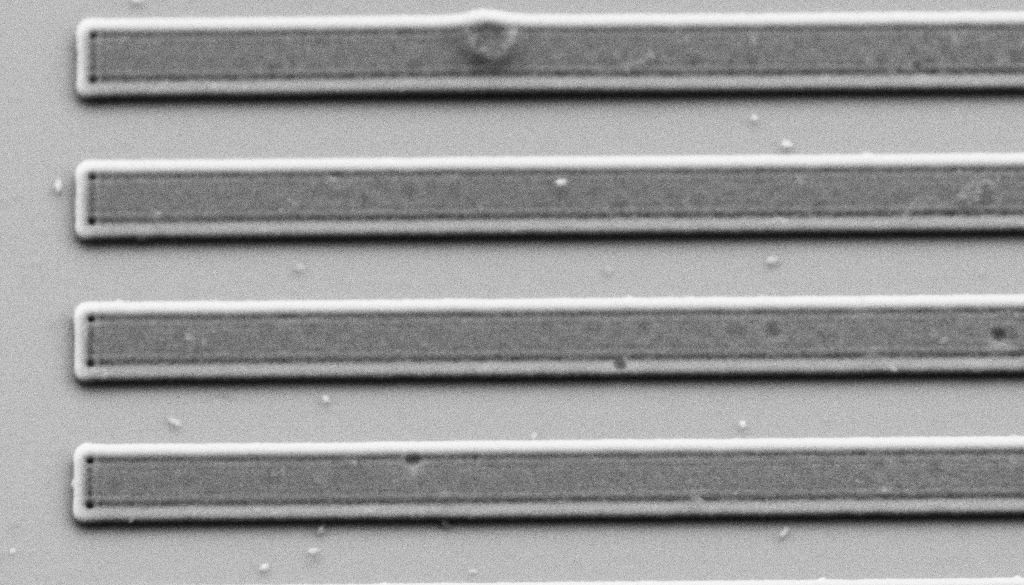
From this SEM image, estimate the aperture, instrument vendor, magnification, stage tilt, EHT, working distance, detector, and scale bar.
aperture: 30 µm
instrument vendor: Zeiss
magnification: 29.55 K X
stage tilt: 30°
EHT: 3 kV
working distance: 5 mm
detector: SE2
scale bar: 1000 nm